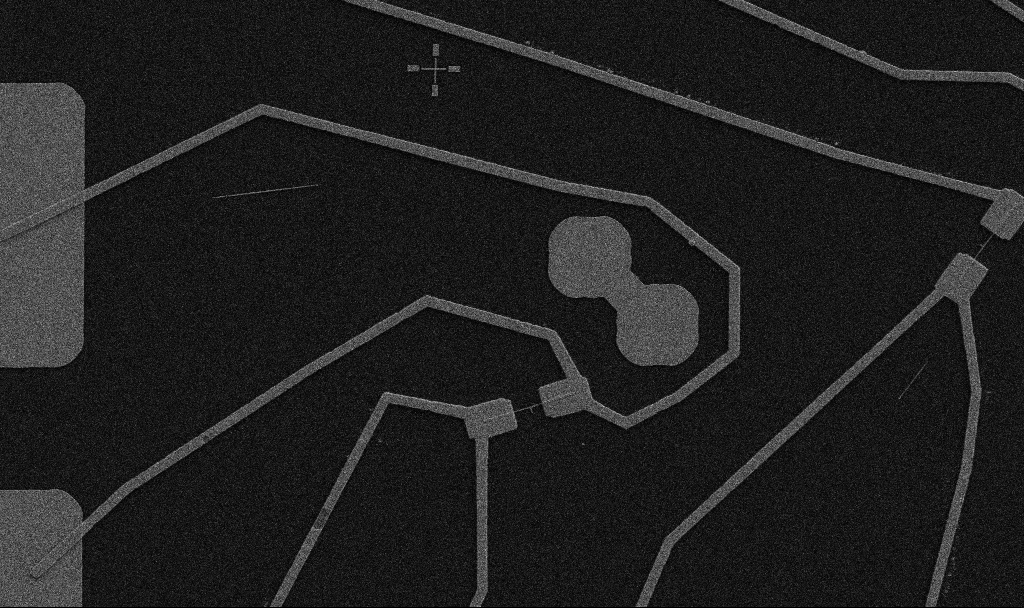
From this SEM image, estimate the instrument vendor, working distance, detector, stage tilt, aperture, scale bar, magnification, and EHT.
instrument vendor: Zeiss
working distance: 10.7 mm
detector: SE2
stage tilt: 0°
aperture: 30 µm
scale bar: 10000 nm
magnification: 5 K X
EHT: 5 kV